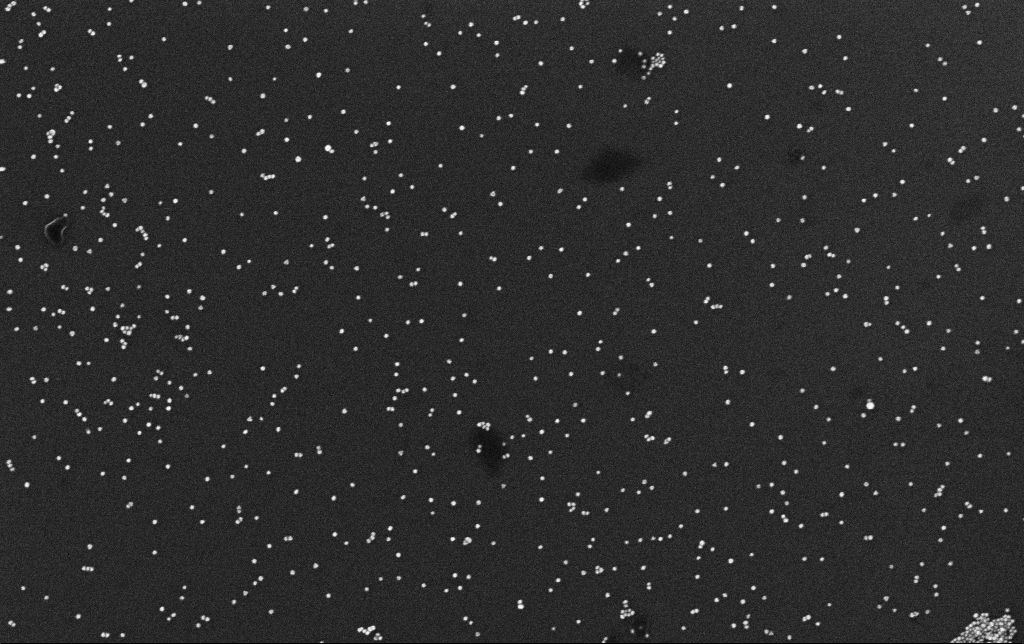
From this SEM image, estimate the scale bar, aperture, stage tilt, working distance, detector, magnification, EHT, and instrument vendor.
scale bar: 200 nm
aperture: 30 µm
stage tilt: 0°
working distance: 3.1 mm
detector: InLens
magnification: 100 K X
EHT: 10 kV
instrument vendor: Zeiss